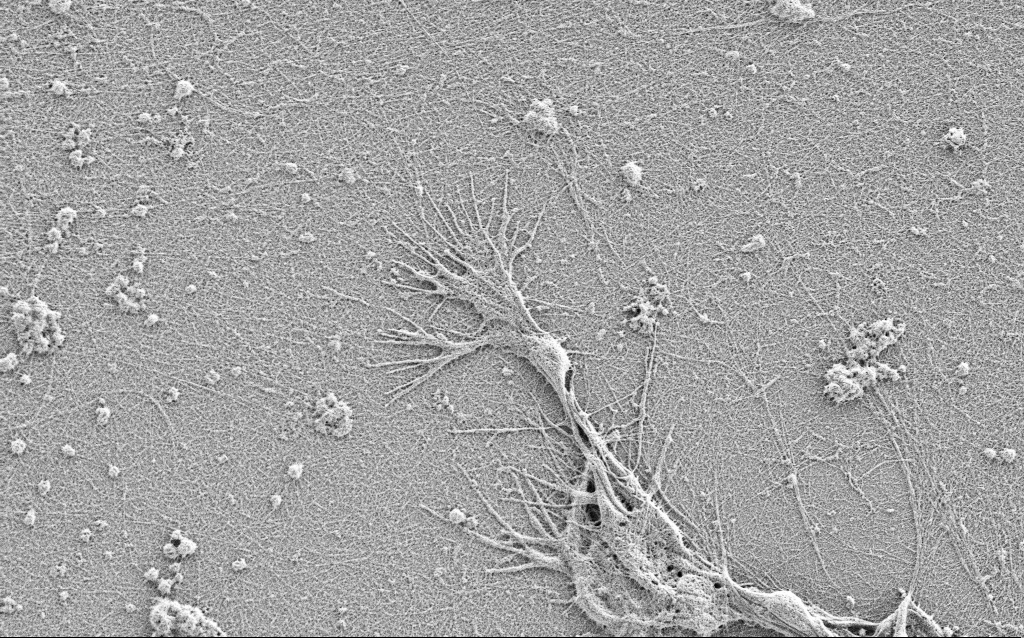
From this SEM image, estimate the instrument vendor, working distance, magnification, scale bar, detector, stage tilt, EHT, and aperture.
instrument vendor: Zeiss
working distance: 3 mm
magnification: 7.5 K X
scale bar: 2000 nm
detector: SE2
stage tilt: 0°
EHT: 0.9 kV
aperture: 30 µm